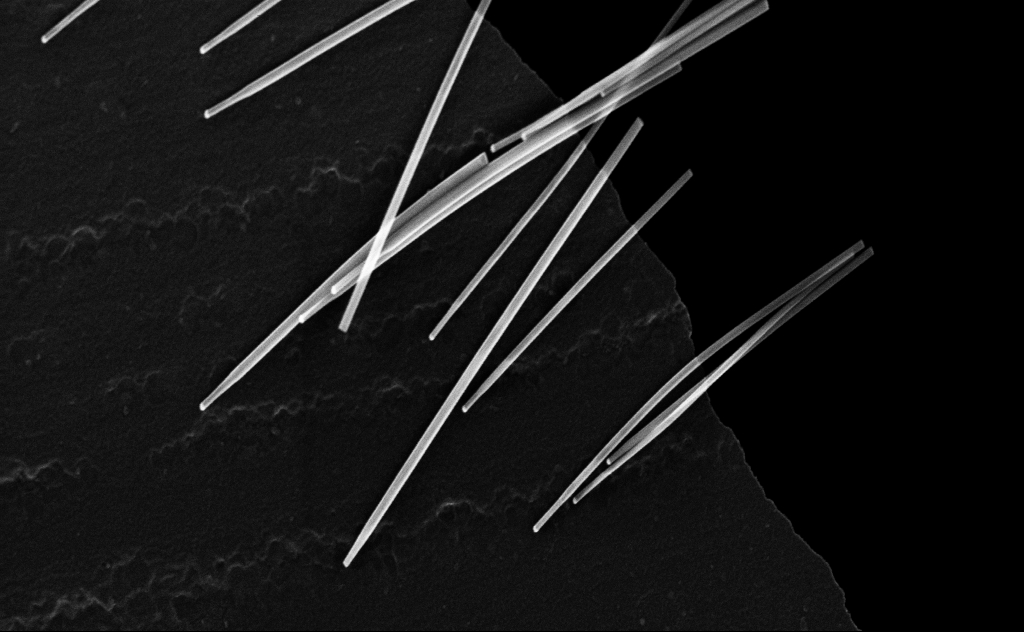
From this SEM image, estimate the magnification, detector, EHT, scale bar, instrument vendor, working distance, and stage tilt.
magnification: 88.44 K X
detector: InLens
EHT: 20 kV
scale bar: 200 nm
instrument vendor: Zeiss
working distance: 8 mm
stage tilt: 0°